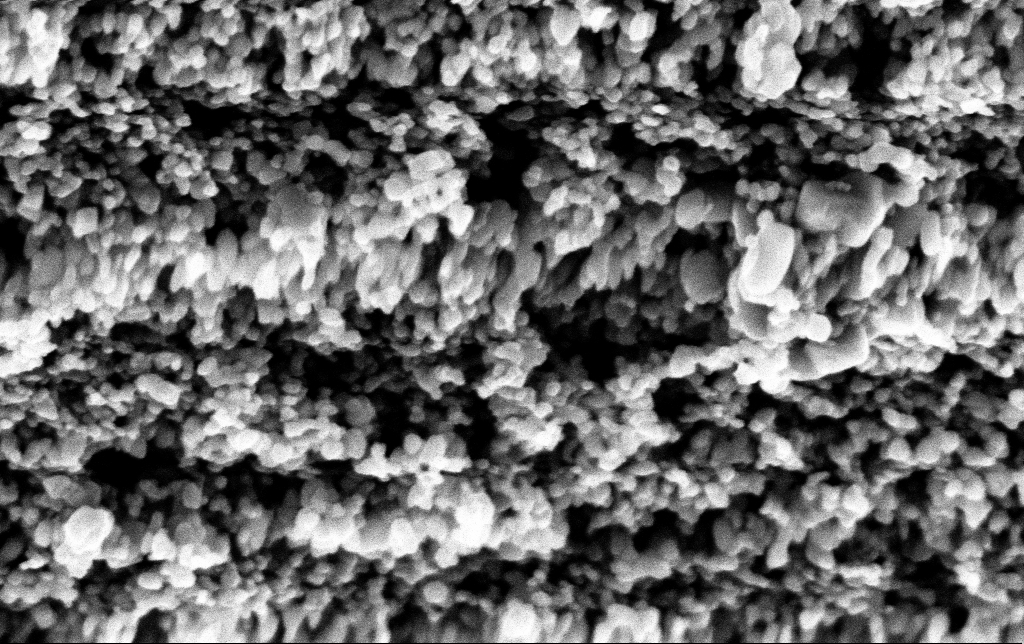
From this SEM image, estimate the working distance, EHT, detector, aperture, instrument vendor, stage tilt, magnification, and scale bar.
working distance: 2.8 mm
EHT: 3 kV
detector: InLens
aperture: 30 µm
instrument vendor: Zeiss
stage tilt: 0°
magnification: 196.98 K X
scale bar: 200 nm